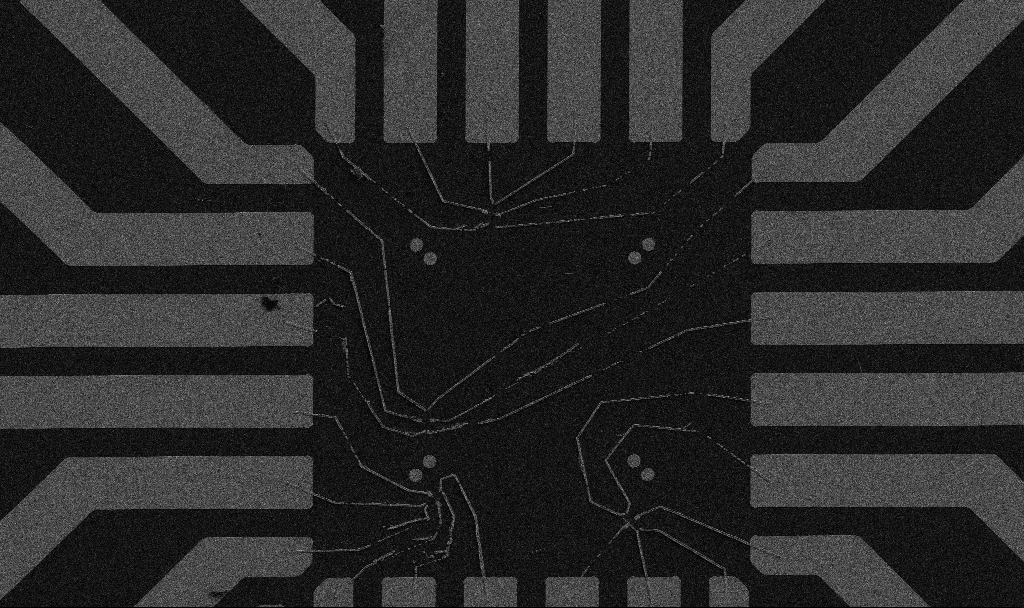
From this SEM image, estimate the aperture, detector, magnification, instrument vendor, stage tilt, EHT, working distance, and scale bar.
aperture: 30 µm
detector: SE2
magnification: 1 K X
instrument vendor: Zeiss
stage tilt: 0°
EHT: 5 kV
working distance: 10.7 mm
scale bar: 20000 nm